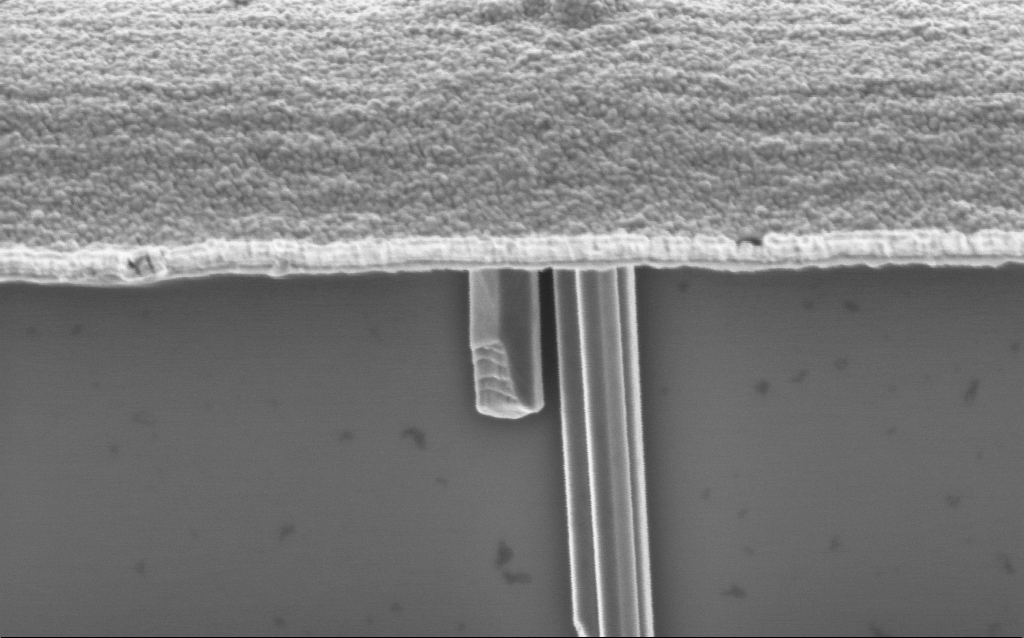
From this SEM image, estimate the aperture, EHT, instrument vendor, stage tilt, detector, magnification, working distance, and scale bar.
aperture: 30 µm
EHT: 2 kV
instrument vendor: Zeiss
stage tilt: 0°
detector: InLens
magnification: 90.08 K X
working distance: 7.7 mm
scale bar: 200 nm